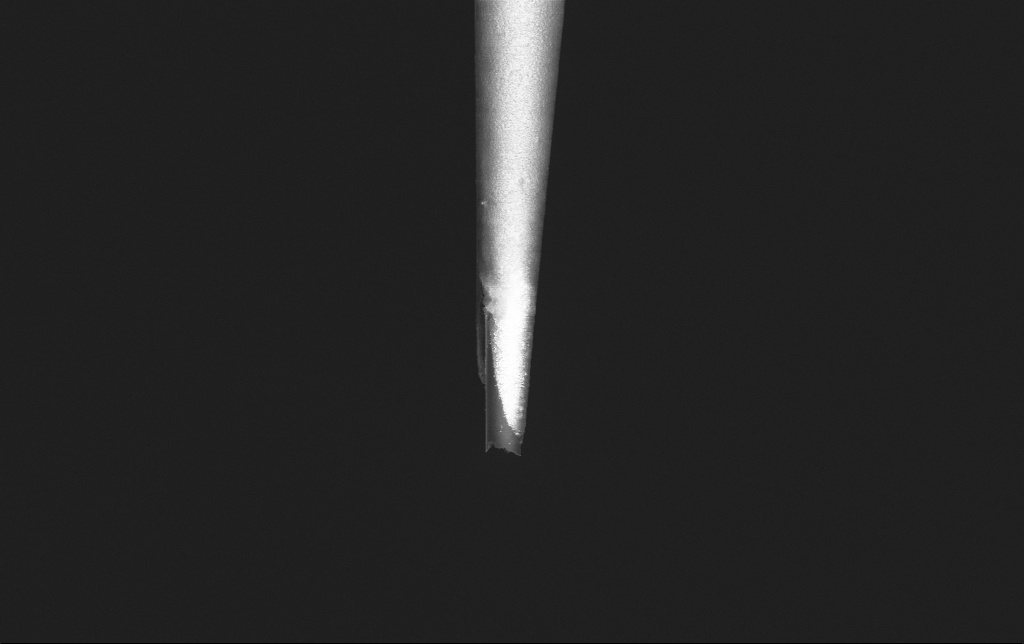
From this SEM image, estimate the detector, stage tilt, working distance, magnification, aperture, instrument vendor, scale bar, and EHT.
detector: InLens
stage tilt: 0°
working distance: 5.9 mm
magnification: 10 K X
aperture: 30 µm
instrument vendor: Zeiss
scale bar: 2000 nm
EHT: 2 kV